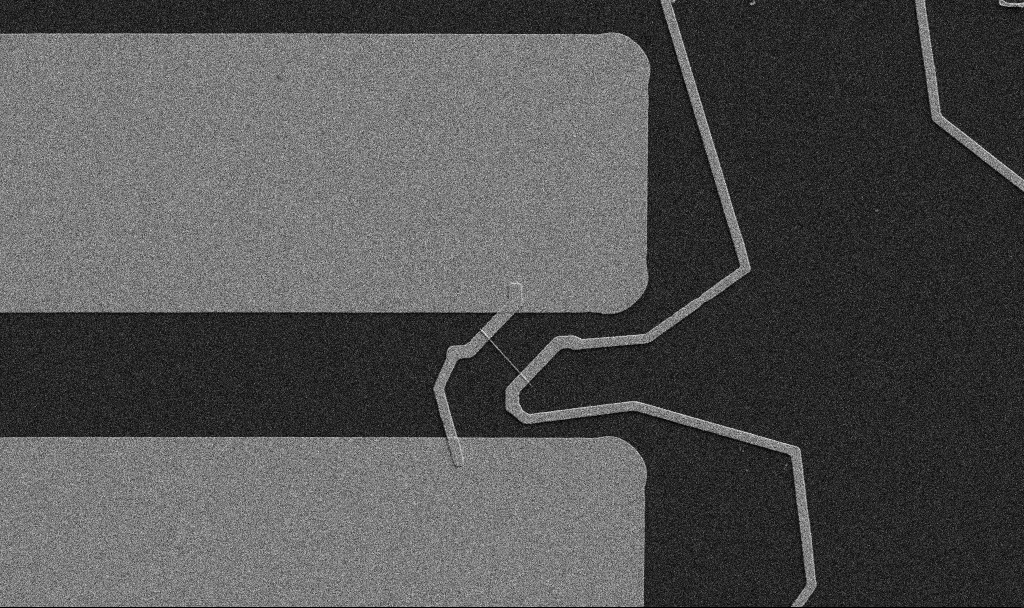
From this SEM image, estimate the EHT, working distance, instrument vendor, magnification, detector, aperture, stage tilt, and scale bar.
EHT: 5 kV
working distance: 10.7 mm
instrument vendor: Zeiss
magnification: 5 K X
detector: SE2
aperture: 30 µm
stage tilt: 0°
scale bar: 10000 nm